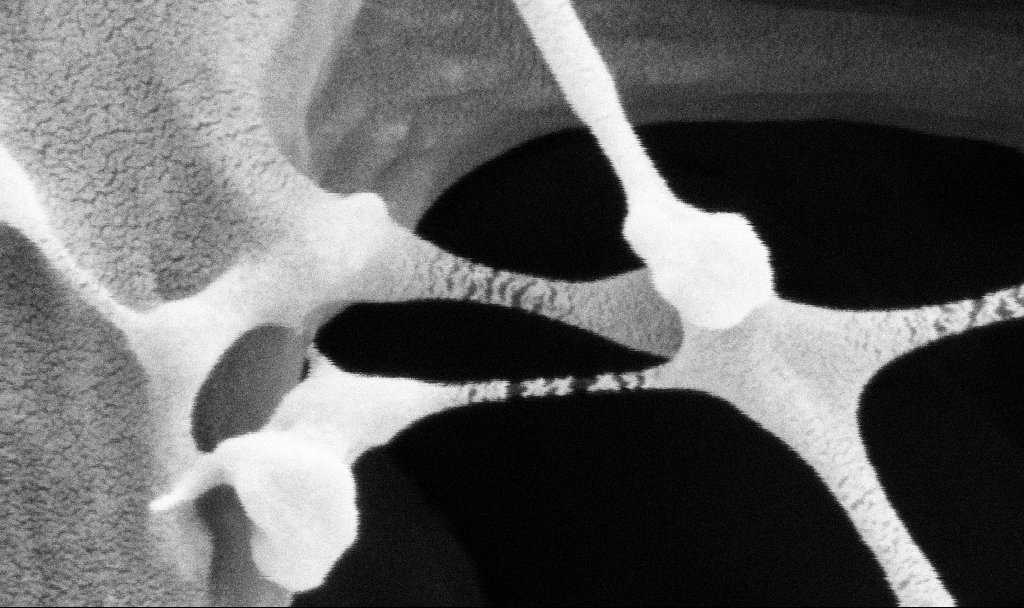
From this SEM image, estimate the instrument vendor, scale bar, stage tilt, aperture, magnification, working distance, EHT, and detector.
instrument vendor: Zeiss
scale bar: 200 nm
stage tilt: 0°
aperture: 30 µm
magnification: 300 K X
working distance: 3.7 mm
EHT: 2 kV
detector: SE2